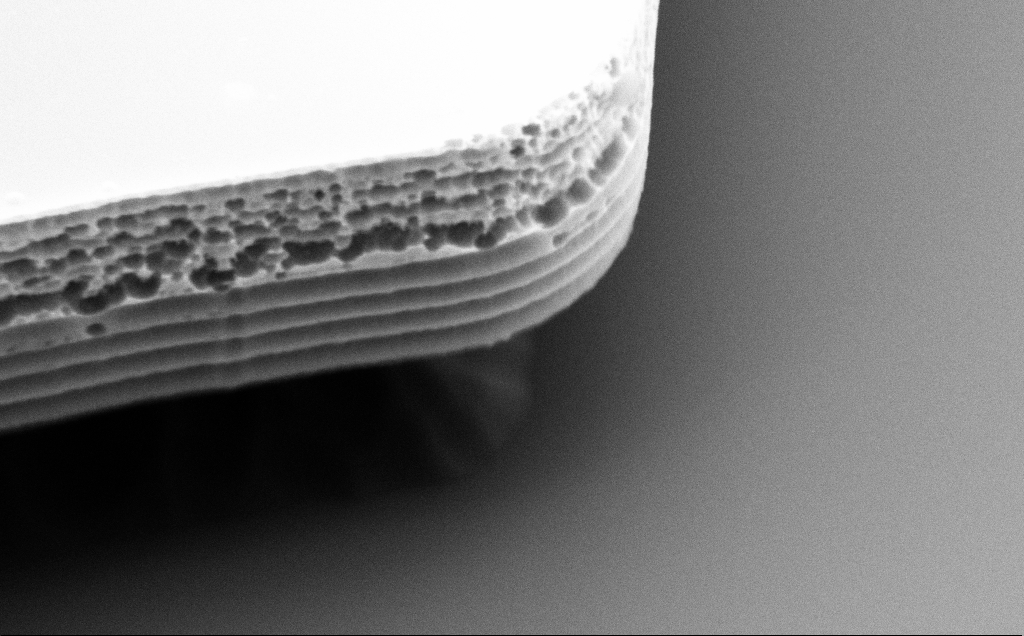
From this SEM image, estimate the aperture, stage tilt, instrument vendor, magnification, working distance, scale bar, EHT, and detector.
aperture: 30 µm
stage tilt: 50°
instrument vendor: Zeiss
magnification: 43.41 K X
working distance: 10 mm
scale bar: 1000 nm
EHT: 5 kV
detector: SE2